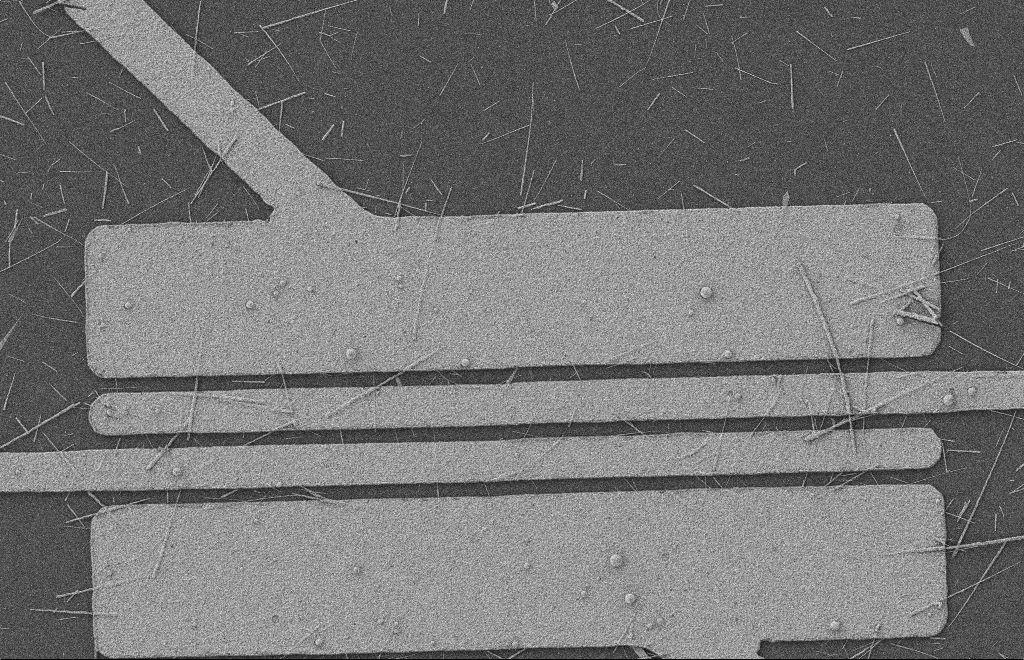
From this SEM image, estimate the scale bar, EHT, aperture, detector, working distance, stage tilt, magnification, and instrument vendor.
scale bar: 2000 nm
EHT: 2 kV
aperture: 20 µm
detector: SE2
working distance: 8 mm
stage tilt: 0°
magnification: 5.13 K X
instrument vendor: Zeiss